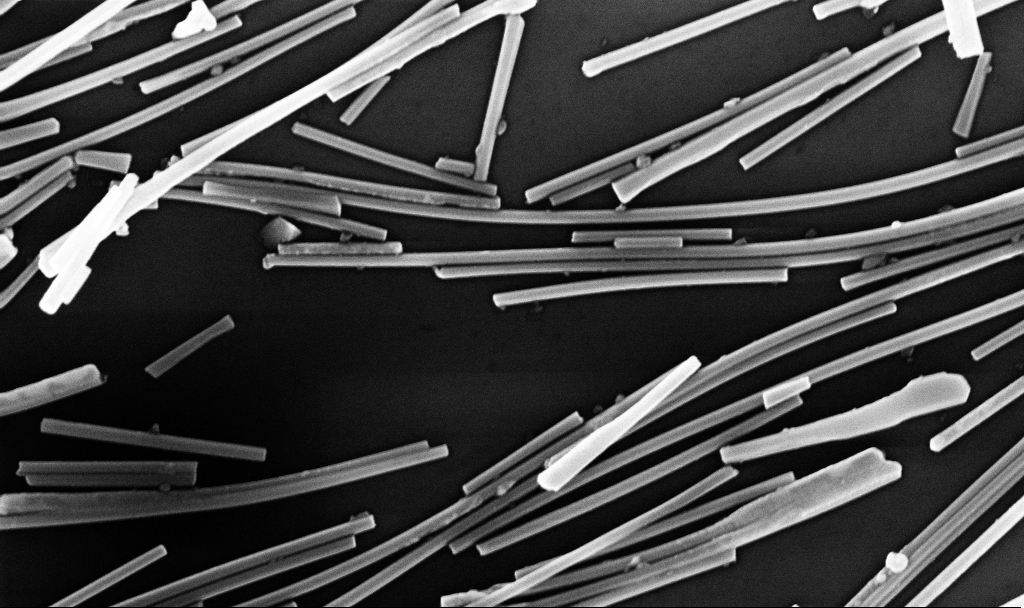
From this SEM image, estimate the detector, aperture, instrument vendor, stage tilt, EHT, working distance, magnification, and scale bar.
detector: InLens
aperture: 30 µm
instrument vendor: Zeiss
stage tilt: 0°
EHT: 10 kV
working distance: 6.7 mm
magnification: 44.93 K X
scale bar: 1000 nm